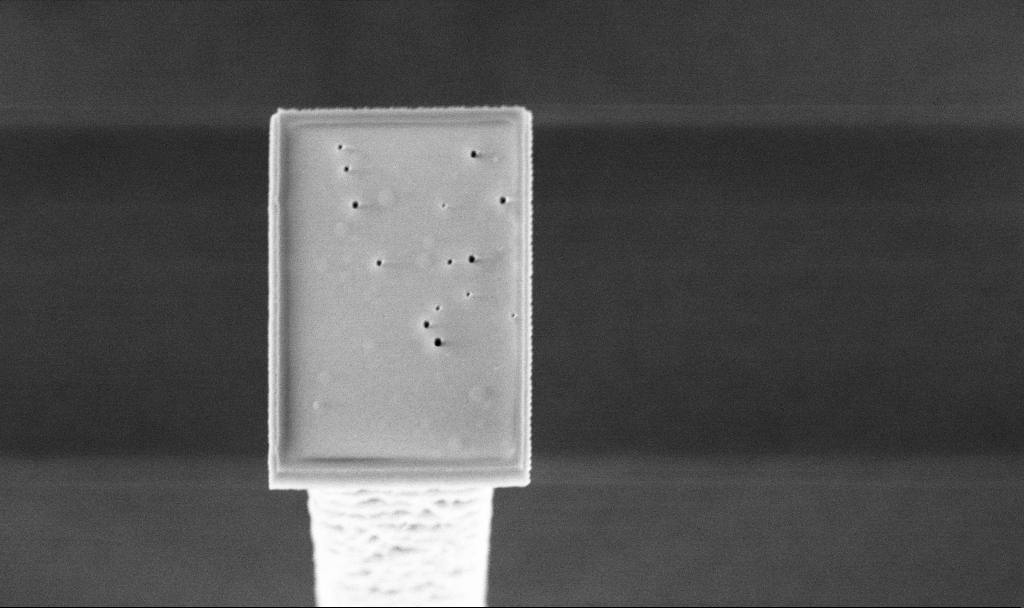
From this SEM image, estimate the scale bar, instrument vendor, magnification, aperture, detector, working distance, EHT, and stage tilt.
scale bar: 2000 nm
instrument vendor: Zeiss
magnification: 32.73 K X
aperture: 30 µm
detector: InLens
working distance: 4.7 mm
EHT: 5 kV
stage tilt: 20°